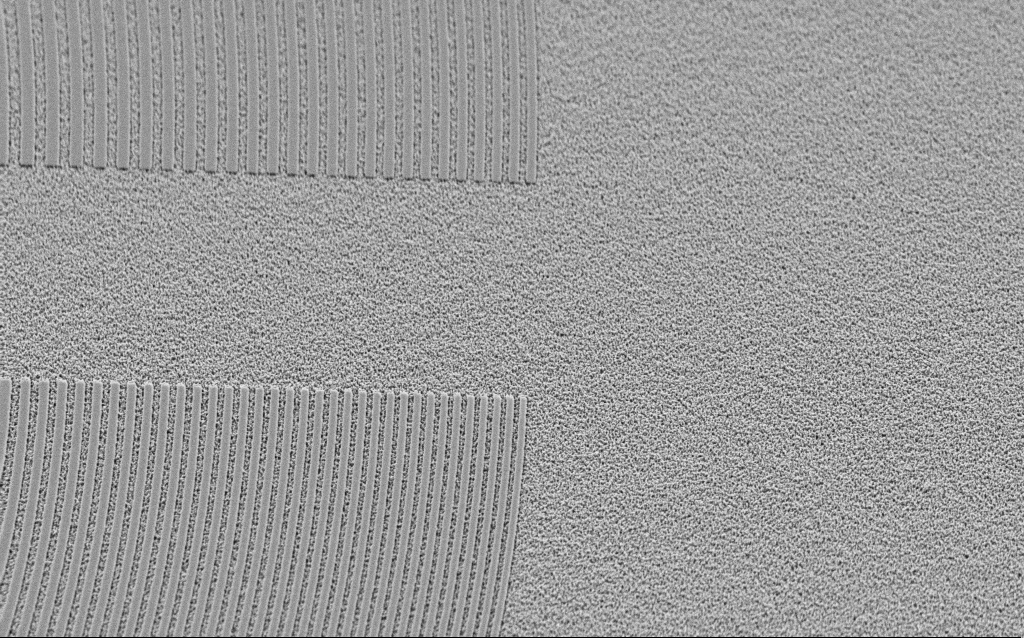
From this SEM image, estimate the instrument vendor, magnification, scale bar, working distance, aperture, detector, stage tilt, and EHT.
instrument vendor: Zeiss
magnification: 7.89 K X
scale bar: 2000 nm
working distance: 8 mm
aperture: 30 µm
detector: SE2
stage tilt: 45°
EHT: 3 kV